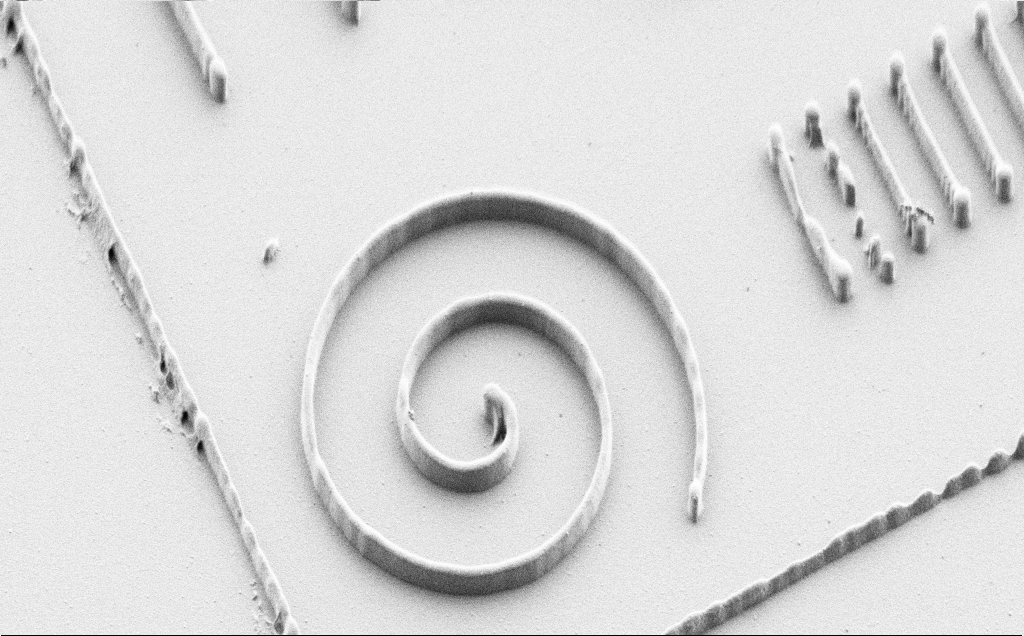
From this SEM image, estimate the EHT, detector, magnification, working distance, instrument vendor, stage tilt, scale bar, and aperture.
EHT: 1 kV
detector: SE2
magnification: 5.48 K X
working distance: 7 mm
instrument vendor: Zeiss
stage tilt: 45°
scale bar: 10000 nm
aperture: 30 µm